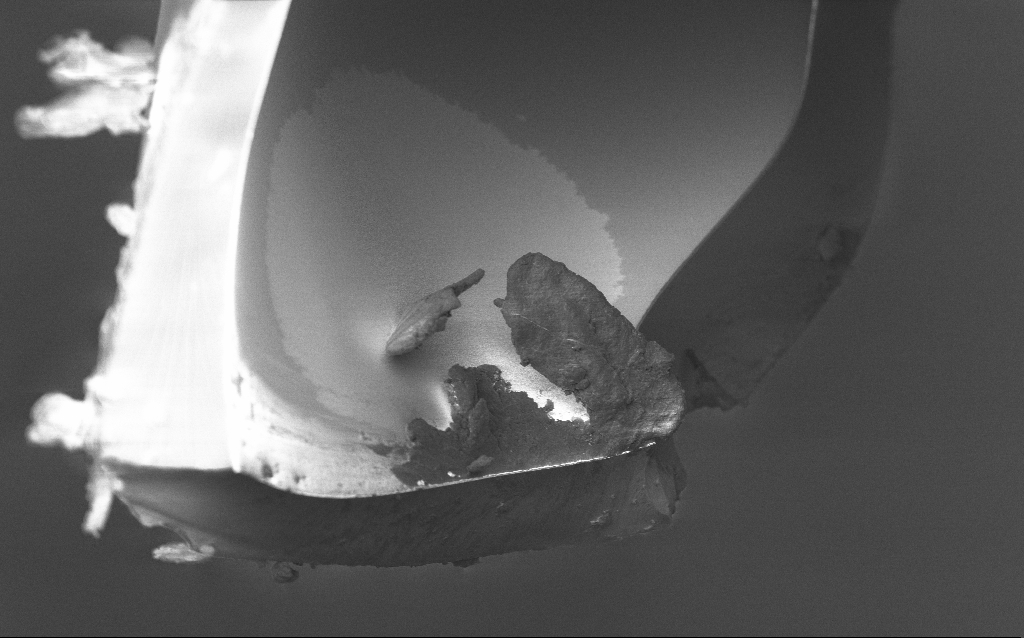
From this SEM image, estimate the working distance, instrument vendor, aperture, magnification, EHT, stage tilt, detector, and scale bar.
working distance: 5 mm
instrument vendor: Zeiss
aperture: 30 µm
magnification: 2.5 K X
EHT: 1 kV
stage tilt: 45°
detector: InLens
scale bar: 20000 nm